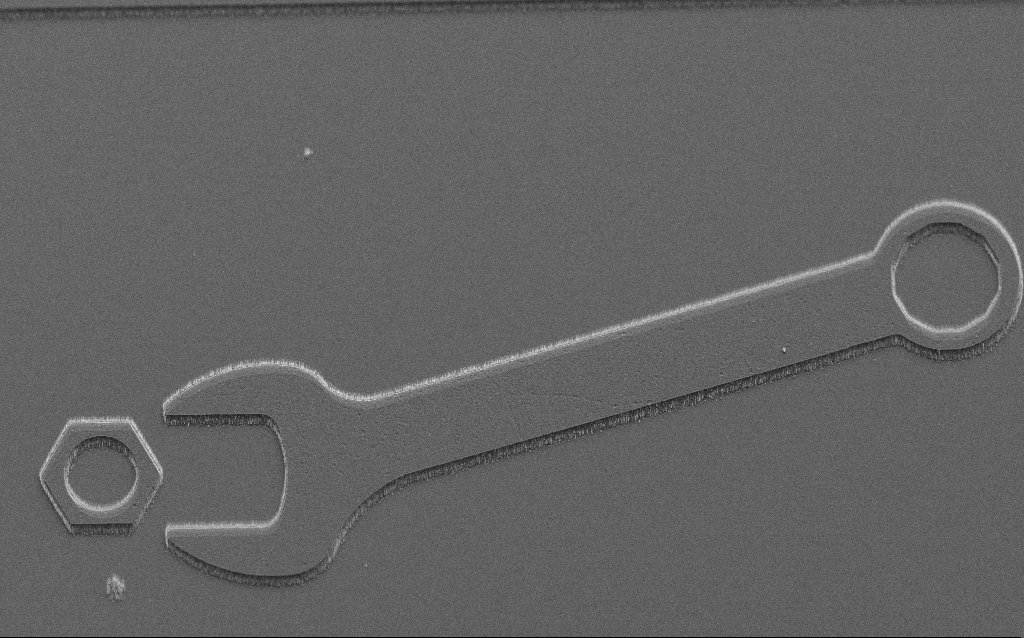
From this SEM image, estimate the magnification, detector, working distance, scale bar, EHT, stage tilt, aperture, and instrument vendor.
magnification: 0.795 K X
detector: SE2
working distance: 8 mm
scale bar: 20000 nm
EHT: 5 kV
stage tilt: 45°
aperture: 30 µm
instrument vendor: Zeiss